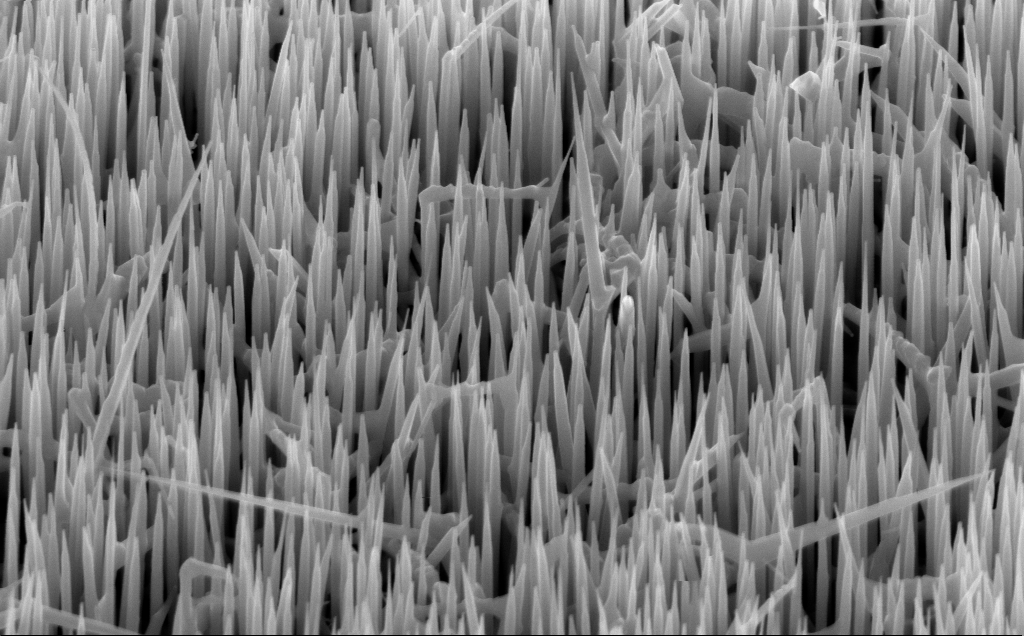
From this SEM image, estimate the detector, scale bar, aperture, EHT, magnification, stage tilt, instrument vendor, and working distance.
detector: InLens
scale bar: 1000 nm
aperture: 30 µm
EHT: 10 kV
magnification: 40 K X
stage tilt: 45°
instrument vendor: Zeiss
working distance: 6 mm